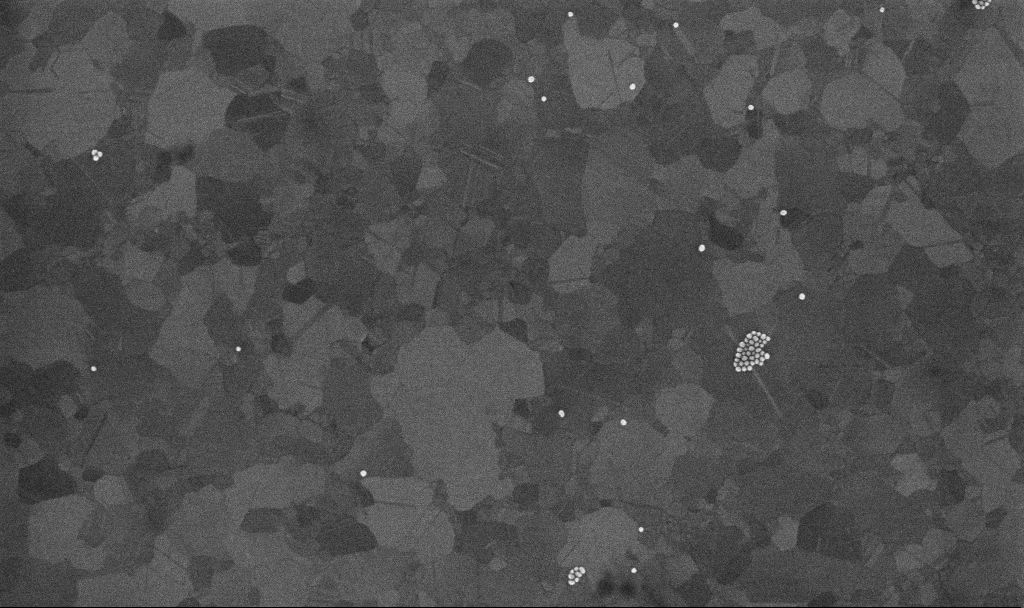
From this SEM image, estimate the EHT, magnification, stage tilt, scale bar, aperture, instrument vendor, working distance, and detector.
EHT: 10 kV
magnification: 100 K X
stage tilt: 0°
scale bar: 200 nm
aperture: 30 µm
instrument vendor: Zeiss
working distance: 3.3 mm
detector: InLens